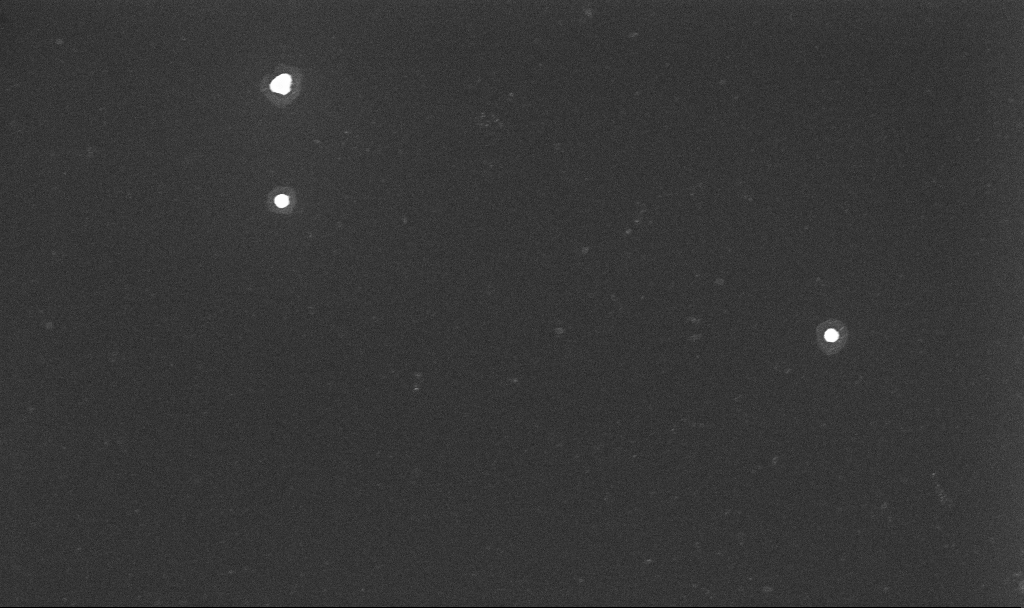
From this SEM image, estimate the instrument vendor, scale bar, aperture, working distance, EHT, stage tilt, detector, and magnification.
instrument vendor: Zeiss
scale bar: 1000 nm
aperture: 30 µm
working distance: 3.3 mm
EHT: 10 kV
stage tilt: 0°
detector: InLens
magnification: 70 K X